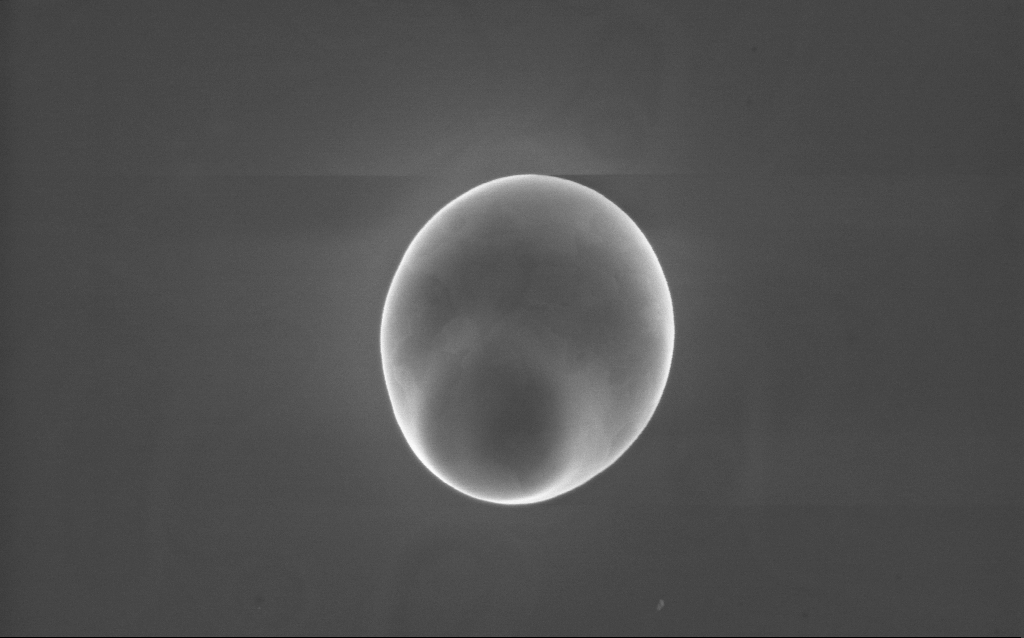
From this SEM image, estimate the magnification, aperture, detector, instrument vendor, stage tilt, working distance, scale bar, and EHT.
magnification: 42 K X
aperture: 30 µm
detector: InLens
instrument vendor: Zeiss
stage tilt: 0°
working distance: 2 mm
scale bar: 1000 nm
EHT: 10 kV